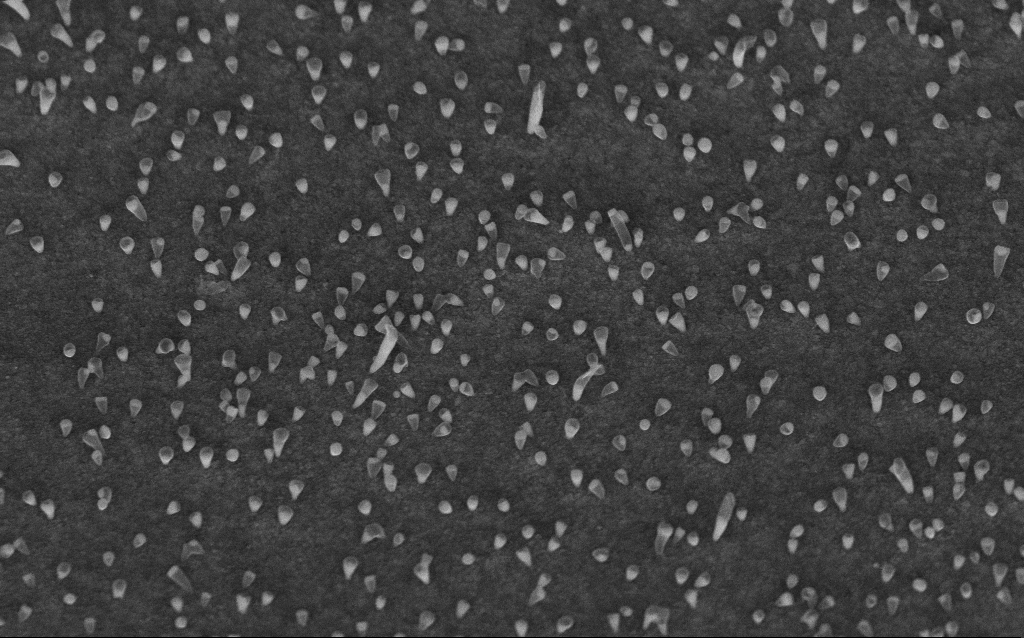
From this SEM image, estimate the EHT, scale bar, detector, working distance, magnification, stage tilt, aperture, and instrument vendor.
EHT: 5 kV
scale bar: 1000 nm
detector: InLens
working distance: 6.6 mm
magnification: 50 K X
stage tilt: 45°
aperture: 30 µm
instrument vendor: Zeiss